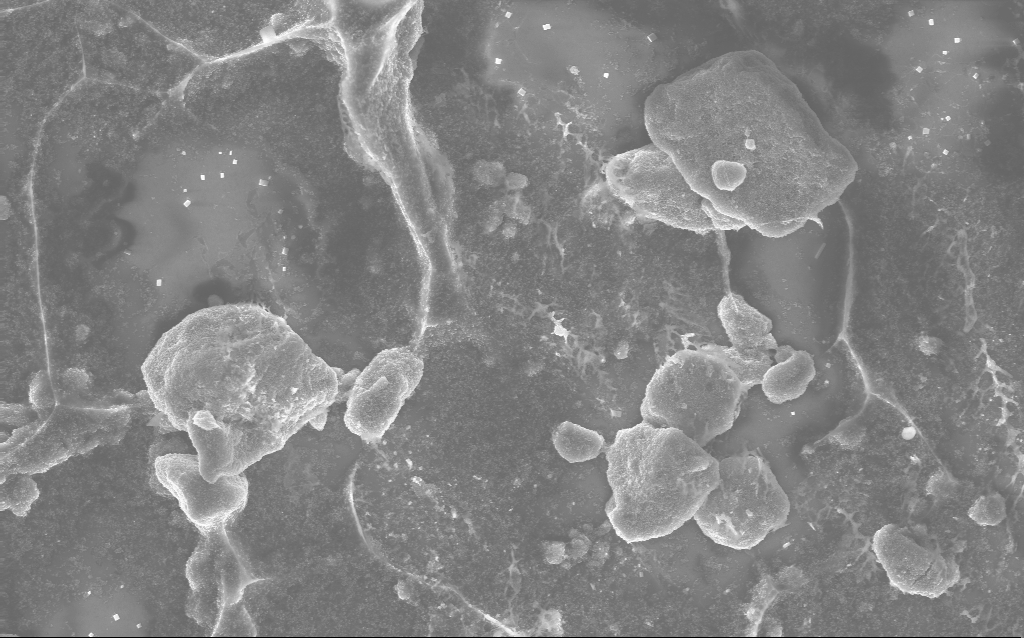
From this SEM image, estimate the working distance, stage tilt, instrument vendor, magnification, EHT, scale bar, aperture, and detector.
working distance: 2.8 mm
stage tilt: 0°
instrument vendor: Zeiss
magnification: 5.83 K X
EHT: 10 kV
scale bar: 10000 nm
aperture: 30 µm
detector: InLens